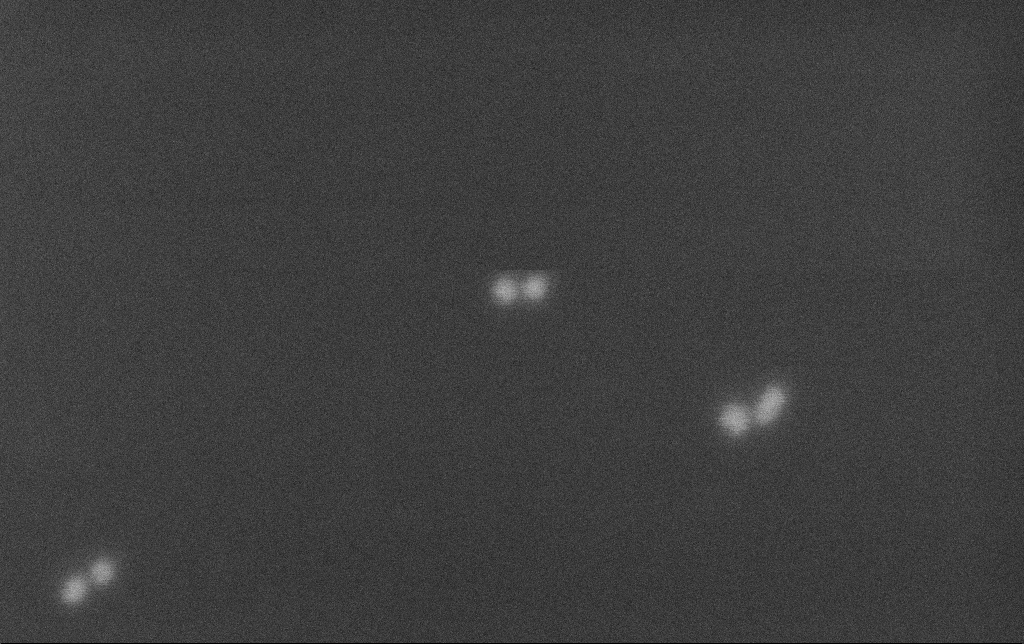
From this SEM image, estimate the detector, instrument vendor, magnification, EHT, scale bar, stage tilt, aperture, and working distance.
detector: SE2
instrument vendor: Zeiss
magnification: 832.15 K X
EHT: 30 kV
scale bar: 20 nm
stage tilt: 0°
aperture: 20 µm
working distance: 10.8 mm